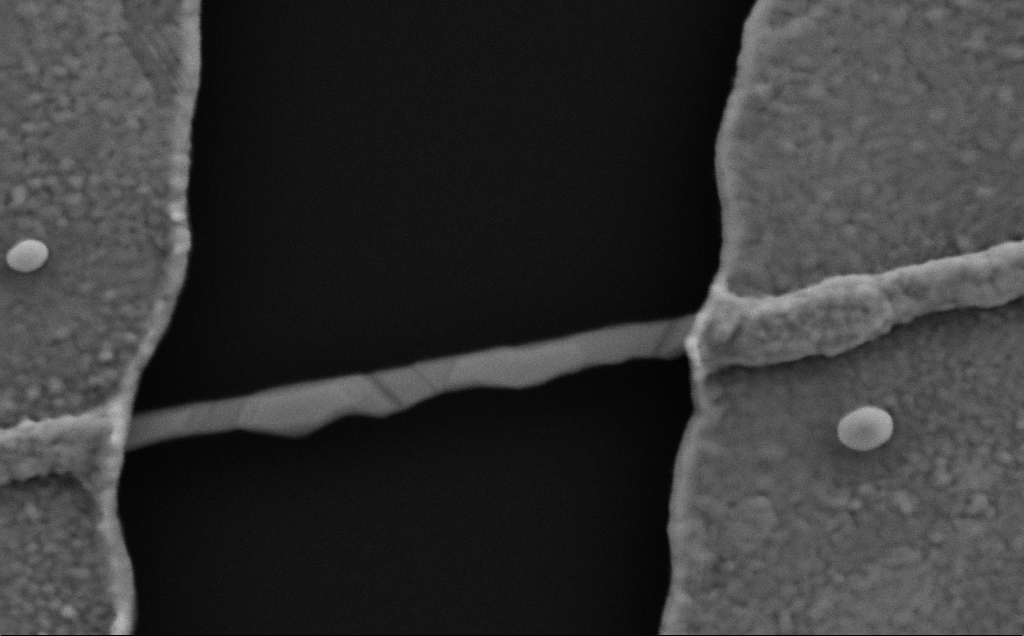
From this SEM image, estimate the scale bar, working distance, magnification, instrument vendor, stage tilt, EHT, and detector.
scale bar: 200 nm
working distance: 6 mm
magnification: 133.17 K X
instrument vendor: Zeiss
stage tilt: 0°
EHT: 5 kV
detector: SE2